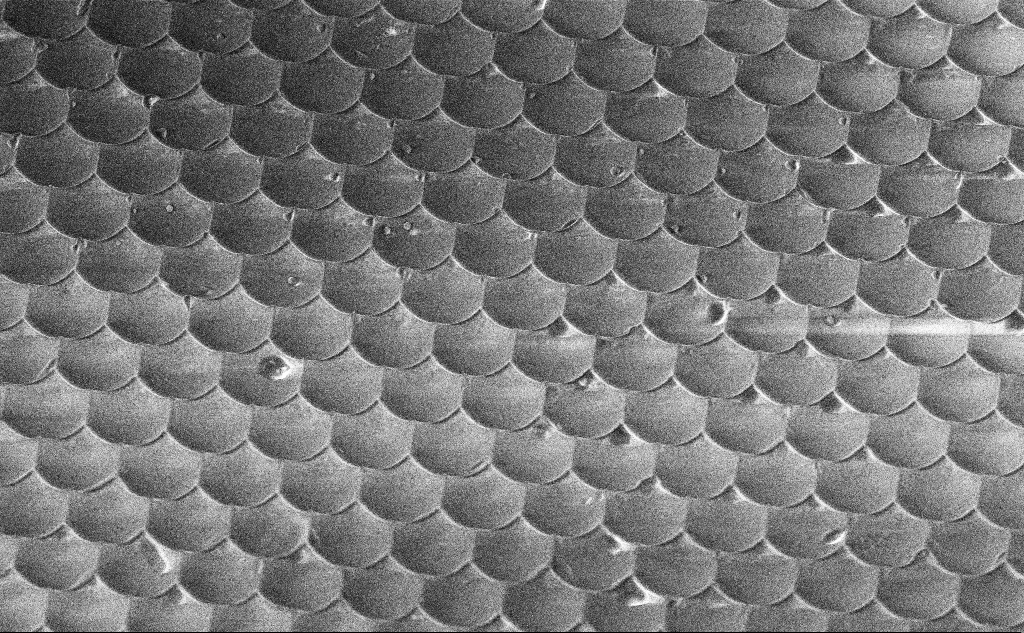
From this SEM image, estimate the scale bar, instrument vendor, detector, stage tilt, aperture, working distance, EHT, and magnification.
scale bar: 2000 nm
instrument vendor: Zeiss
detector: InLens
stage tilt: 45°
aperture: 30 µm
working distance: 10.7 mm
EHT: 3 kV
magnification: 9.9 K X